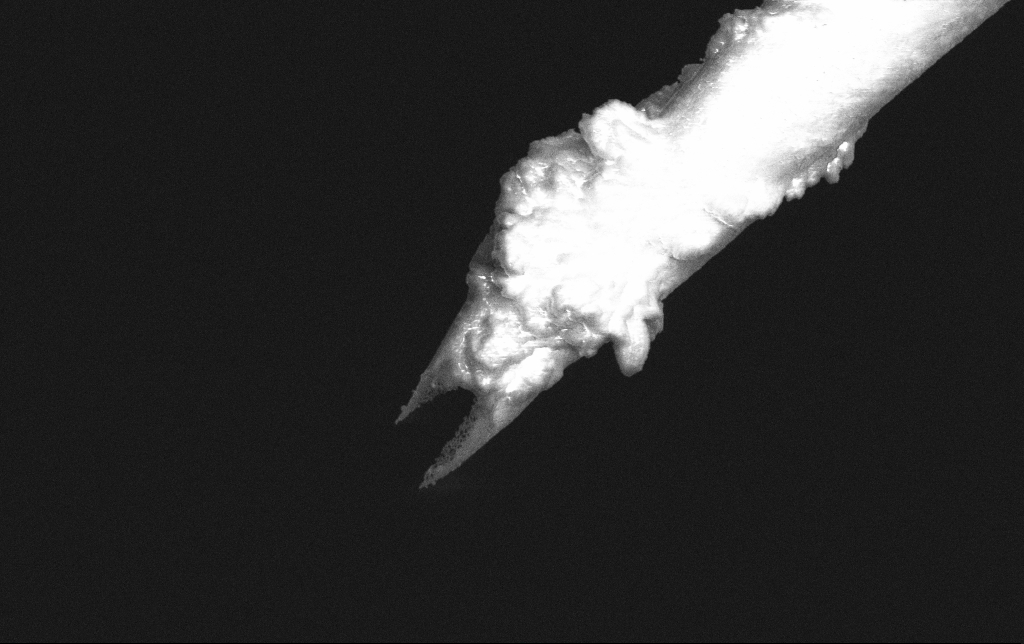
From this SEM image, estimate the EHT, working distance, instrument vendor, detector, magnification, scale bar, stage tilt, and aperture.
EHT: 3 kV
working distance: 7.6 mm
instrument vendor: Zeiss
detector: InLens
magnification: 15 K X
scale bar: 1000 nm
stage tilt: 0°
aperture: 30 µm